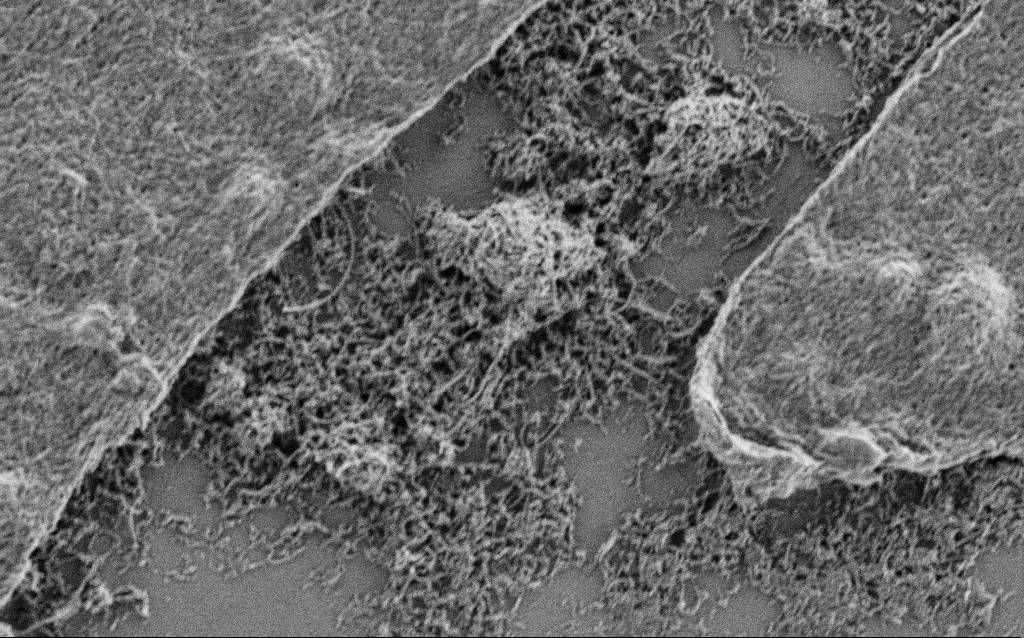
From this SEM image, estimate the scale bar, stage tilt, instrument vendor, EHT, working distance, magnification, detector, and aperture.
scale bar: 1000 nm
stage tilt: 0°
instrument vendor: Zeiss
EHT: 1 kV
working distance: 4 mm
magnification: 20 K X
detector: SE2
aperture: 30 µm